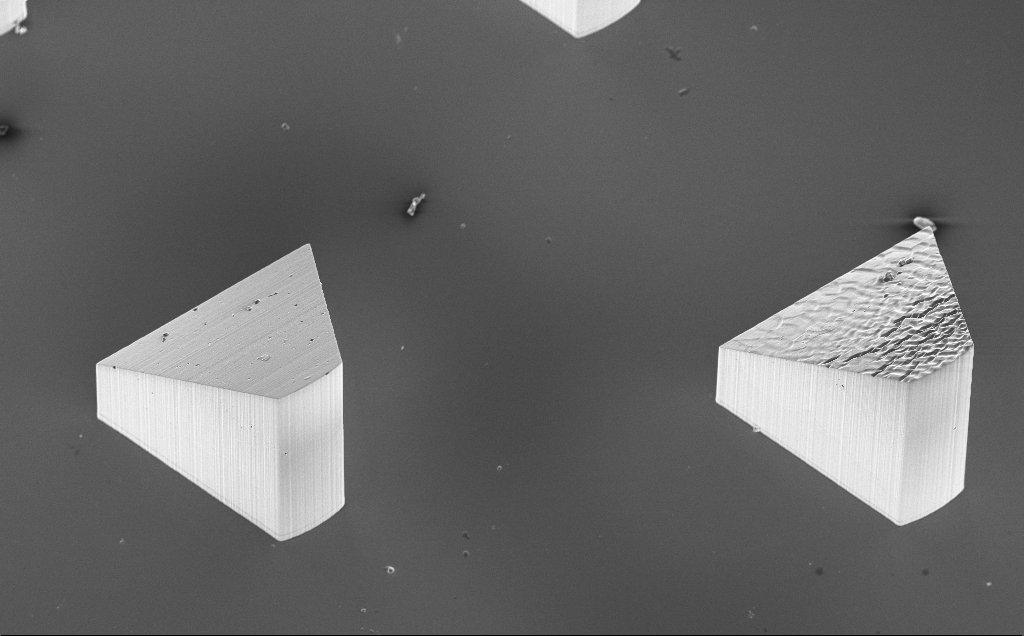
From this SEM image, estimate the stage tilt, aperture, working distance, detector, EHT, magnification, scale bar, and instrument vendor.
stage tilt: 20°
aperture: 30 µm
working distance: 9 mm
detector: InLens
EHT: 10 kV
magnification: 0.231 K X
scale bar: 100000 nm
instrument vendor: Zeiss